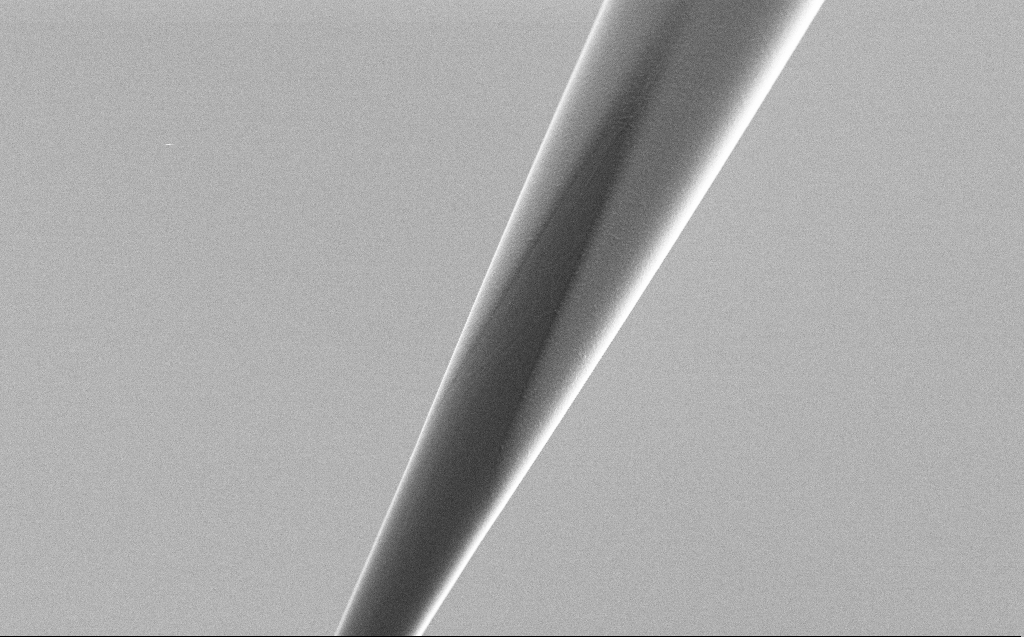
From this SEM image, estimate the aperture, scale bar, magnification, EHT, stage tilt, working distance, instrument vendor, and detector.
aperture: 30 µm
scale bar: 10000 nm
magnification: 5 K X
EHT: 5 kV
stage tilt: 45°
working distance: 6 mm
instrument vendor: Zeiss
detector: SE2